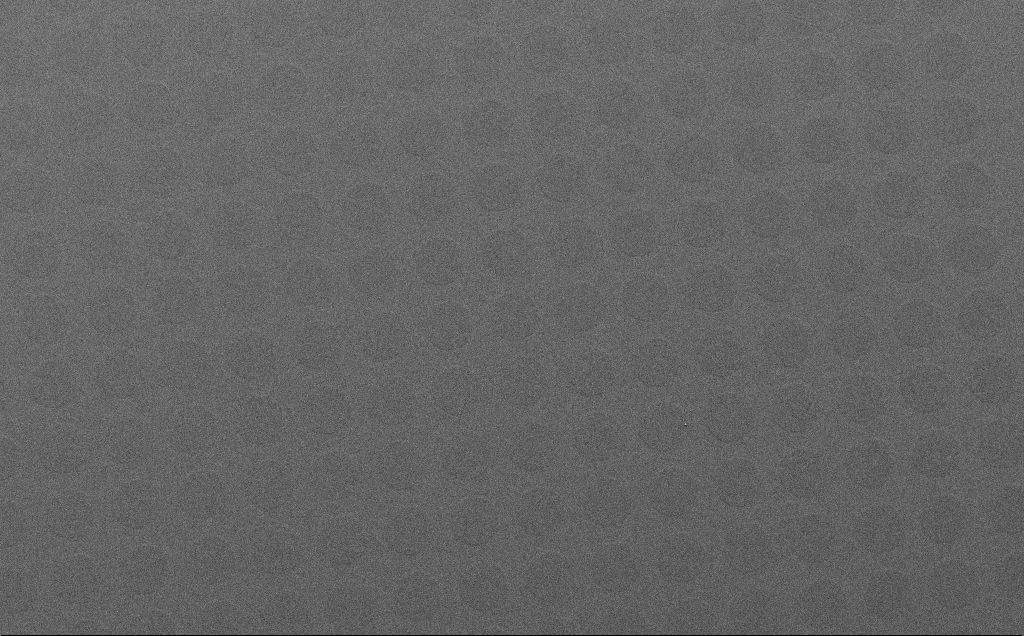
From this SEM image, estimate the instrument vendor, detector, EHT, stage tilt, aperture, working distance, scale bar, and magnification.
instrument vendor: Zeiss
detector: SE2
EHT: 10 kV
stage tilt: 40.4°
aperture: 30 µm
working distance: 6 mm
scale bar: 100000 nm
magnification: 0.501 K X